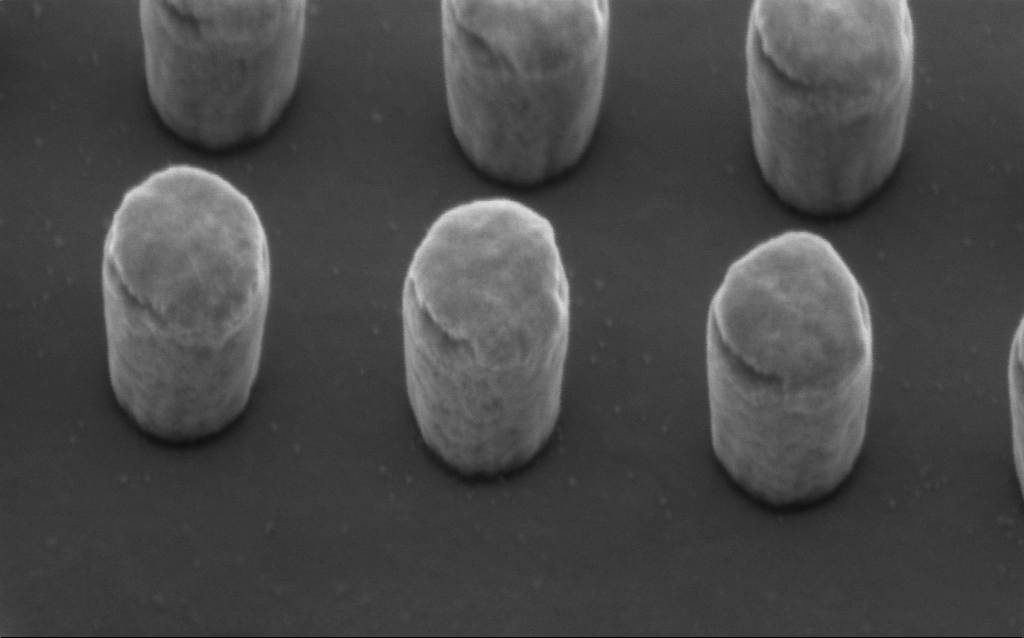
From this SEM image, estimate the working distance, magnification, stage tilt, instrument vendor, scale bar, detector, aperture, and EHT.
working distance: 3 mm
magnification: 225.07 K X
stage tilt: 45°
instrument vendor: Zeiss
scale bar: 200 nm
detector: InLens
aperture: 30 µm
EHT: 2 kV